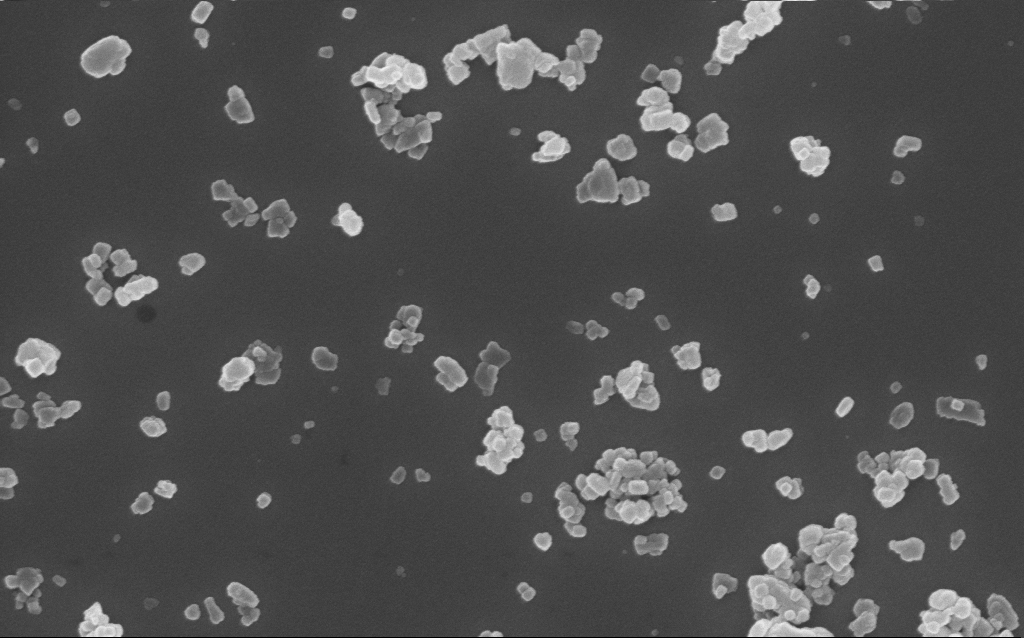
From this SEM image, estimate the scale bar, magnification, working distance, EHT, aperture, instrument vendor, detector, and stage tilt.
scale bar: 1000 nm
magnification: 47.27 K X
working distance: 6 mm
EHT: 10 kV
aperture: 30 µm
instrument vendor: Zeiss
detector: InLens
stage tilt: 0°